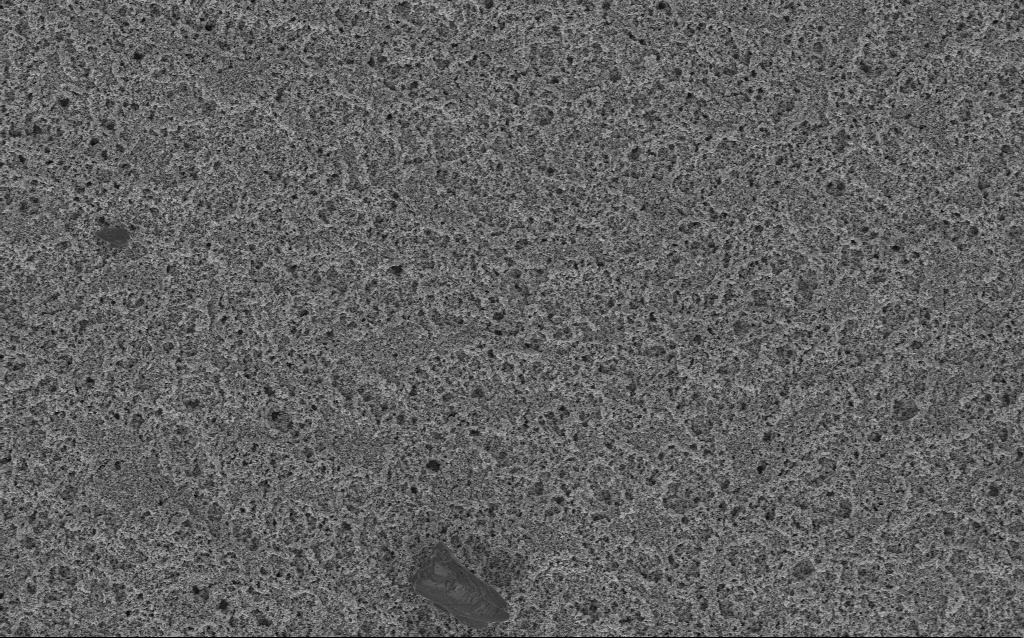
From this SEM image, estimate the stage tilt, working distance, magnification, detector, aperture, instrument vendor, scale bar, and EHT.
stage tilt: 0°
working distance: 10 mm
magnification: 6.61 K X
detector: InLens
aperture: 30 µm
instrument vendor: Zeiss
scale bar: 10000 nm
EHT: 3 kV